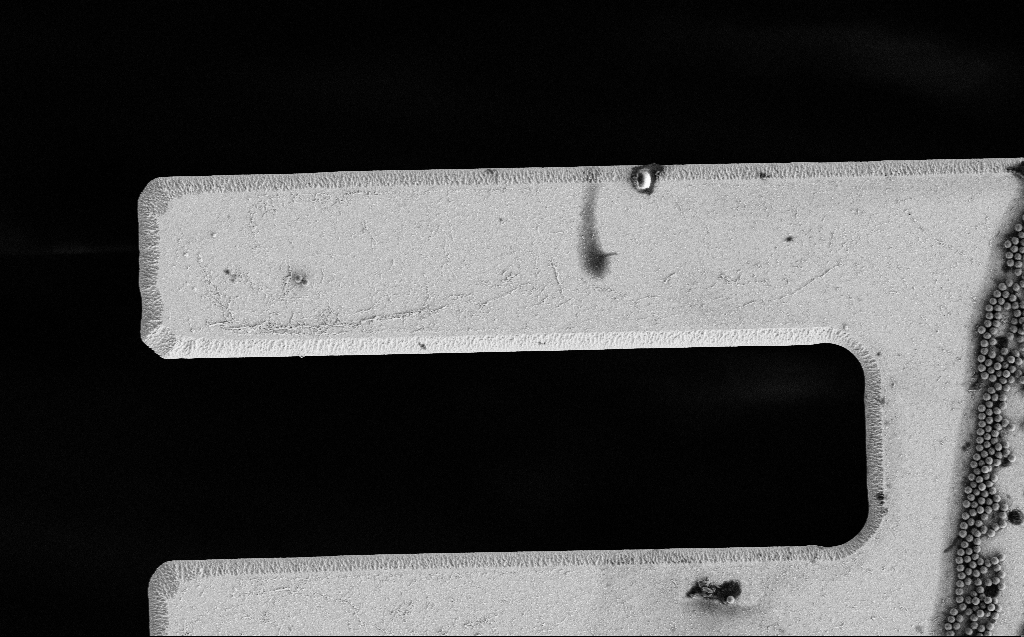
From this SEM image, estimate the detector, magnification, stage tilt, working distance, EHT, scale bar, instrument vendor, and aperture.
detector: SE2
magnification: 3.54 K X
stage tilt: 0°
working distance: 7 mm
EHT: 3 kV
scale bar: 20000 nm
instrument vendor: Zeiss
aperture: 30 µm